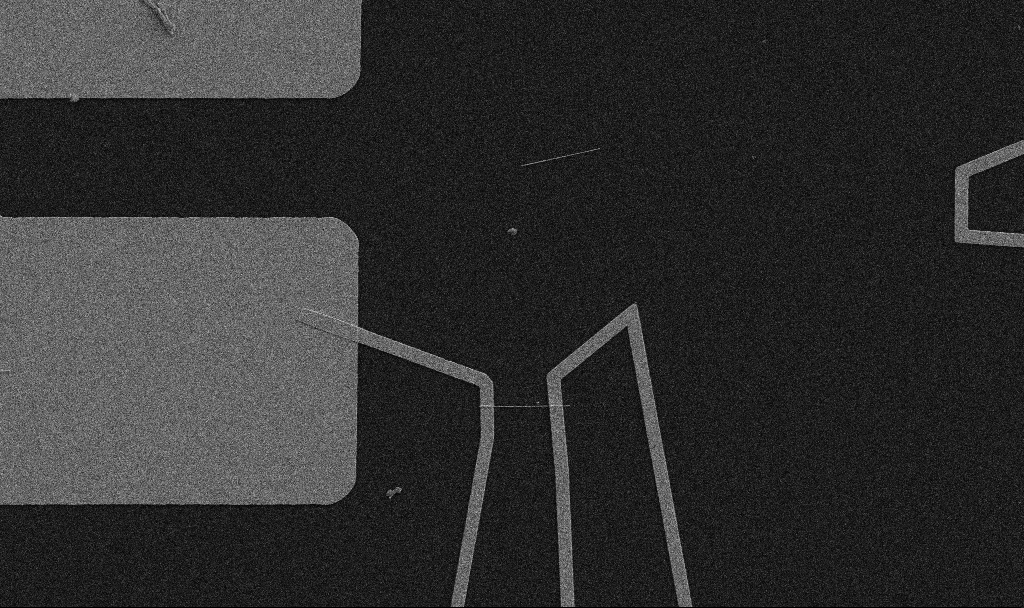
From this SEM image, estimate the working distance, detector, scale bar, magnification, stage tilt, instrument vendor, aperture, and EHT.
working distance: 10.7 mm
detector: SE2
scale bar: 10000 nm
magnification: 5 K X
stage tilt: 0°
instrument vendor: Zeiss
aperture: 30 µm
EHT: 5 kV